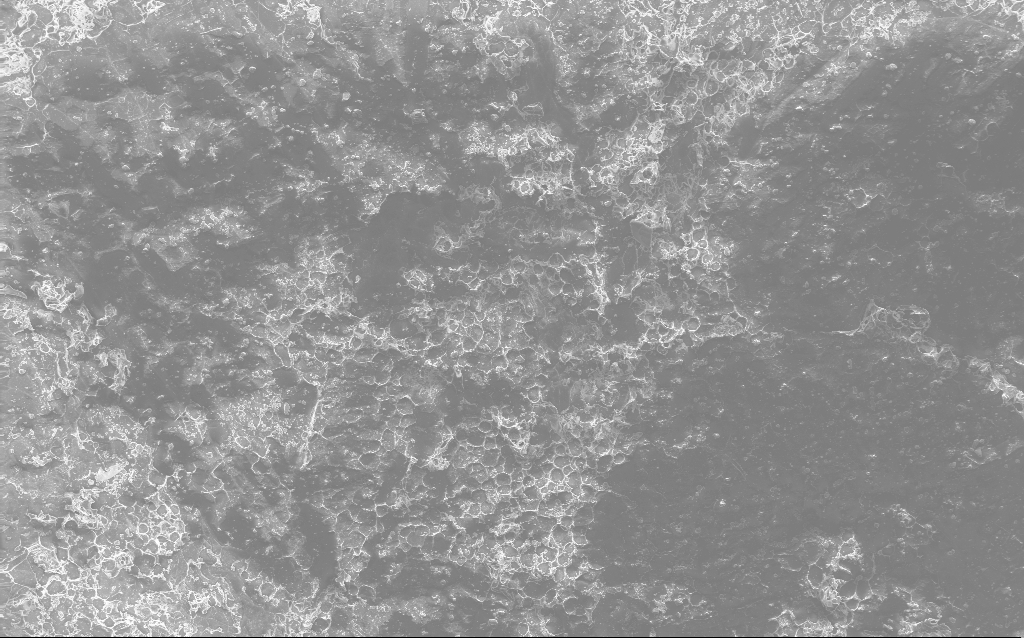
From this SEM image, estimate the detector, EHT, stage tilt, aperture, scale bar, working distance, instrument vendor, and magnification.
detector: InLens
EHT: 10 kV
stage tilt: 0°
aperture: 30 µm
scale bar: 100000 nm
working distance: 2.8 mm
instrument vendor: Zeiss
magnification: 0.235 K X